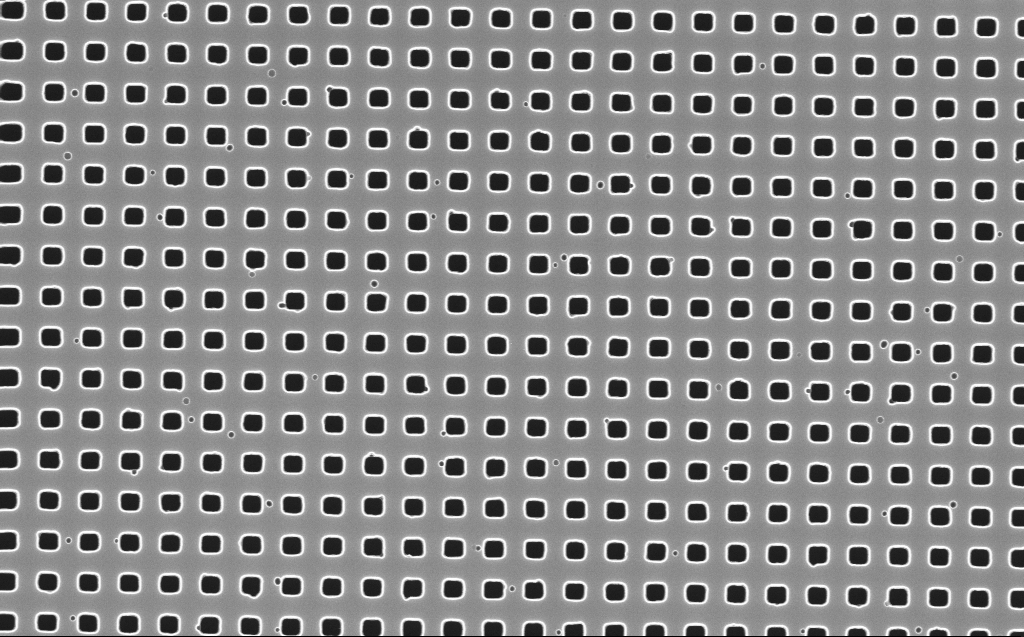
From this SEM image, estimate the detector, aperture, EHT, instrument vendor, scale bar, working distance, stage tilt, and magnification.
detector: InLens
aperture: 30 µm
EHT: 10 kV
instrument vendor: Zeiss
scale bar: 2000 nm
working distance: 6 mm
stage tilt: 0°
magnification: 30 K X